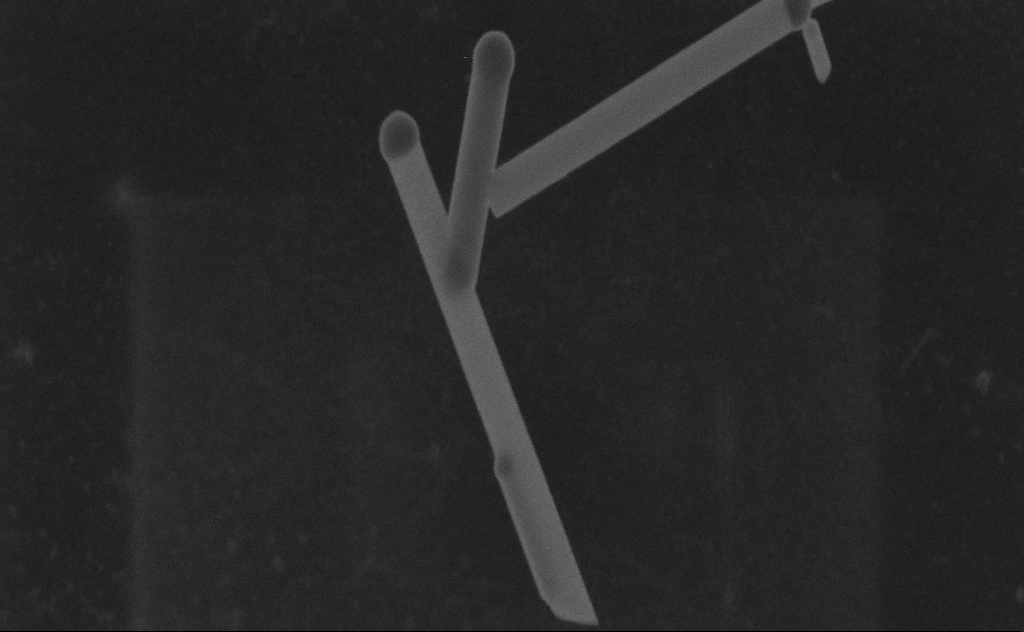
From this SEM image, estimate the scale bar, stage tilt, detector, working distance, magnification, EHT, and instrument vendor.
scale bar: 200 nm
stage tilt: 0°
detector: SE2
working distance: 8 mm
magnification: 181.88 K X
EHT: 20 kV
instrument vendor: Zeiss